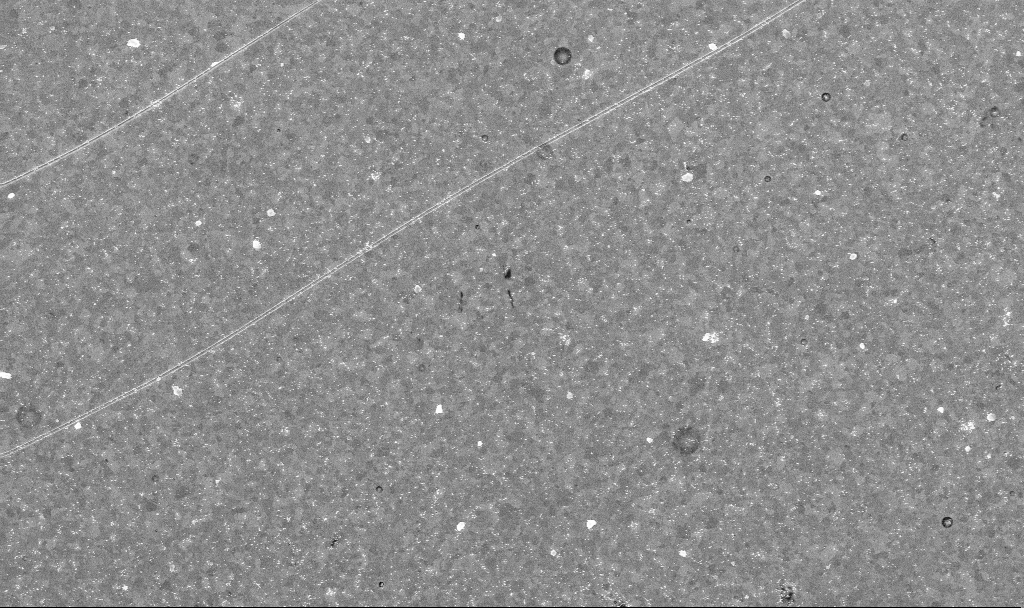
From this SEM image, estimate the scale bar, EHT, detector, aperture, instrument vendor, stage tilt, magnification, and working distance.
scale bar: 2000 nm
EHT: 10 kV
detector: InLens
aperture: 30 µm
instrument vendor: Zeiss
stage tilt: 0°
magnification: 10.04 K X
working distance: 3.4 mm